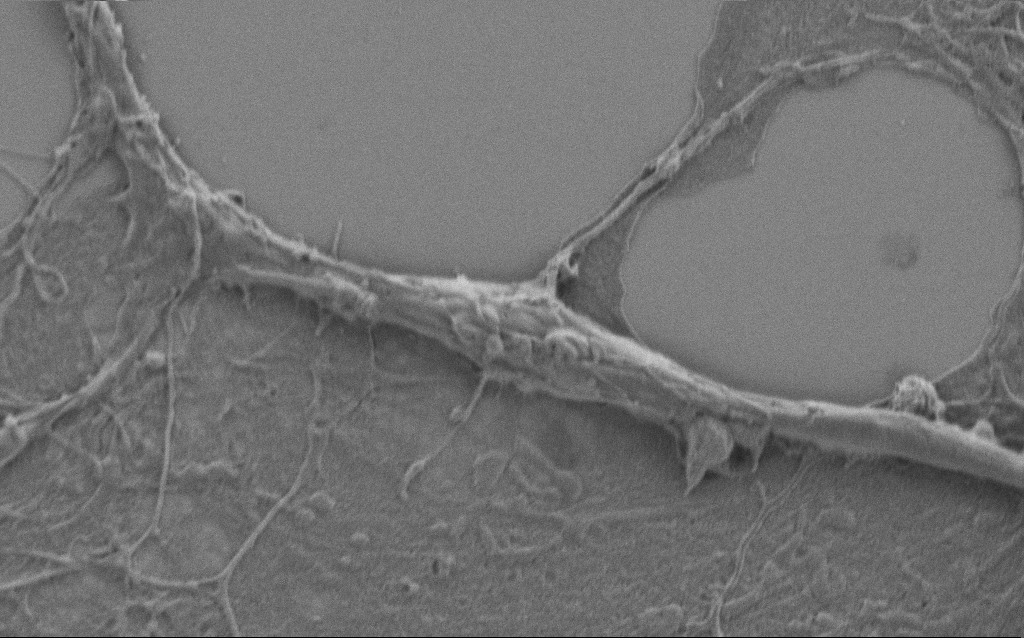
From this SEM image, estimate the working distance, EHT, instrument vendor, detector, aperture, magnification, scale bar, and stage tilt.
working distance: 5.9 mm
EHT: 1 kV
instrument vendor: Zeiss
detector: SE2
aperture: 30 µm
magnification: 15 K X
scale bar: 2000 nm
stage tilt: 0°